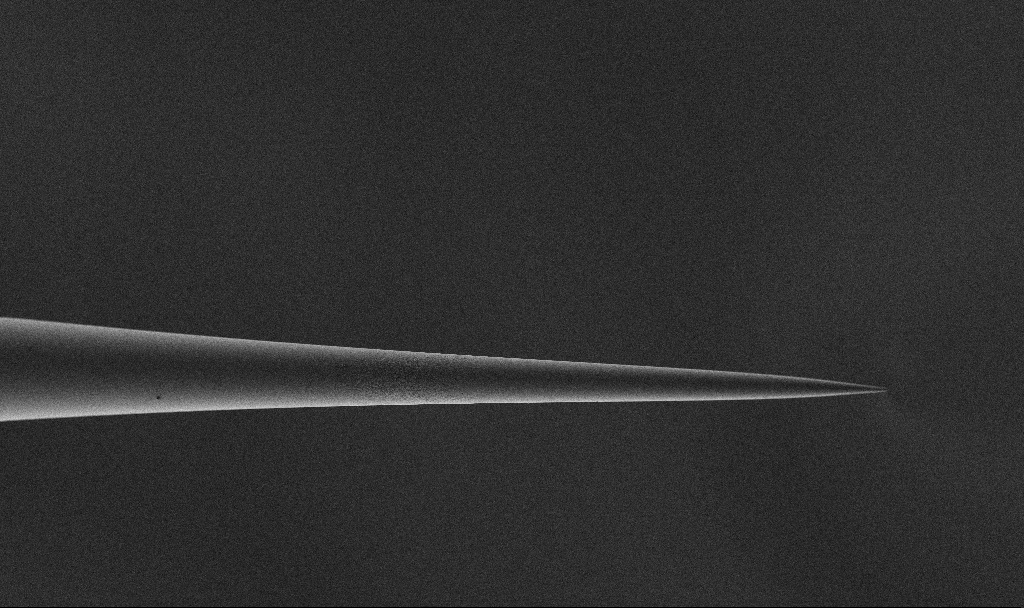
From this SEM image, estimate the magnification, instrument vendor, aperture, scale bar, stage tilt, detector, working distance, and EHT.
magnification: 1.34 K X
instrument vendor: Zeiss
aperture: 30 µm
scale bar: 10000 nm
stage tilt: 45°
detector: SE2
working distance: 3.3 mm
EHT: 1.5 kV